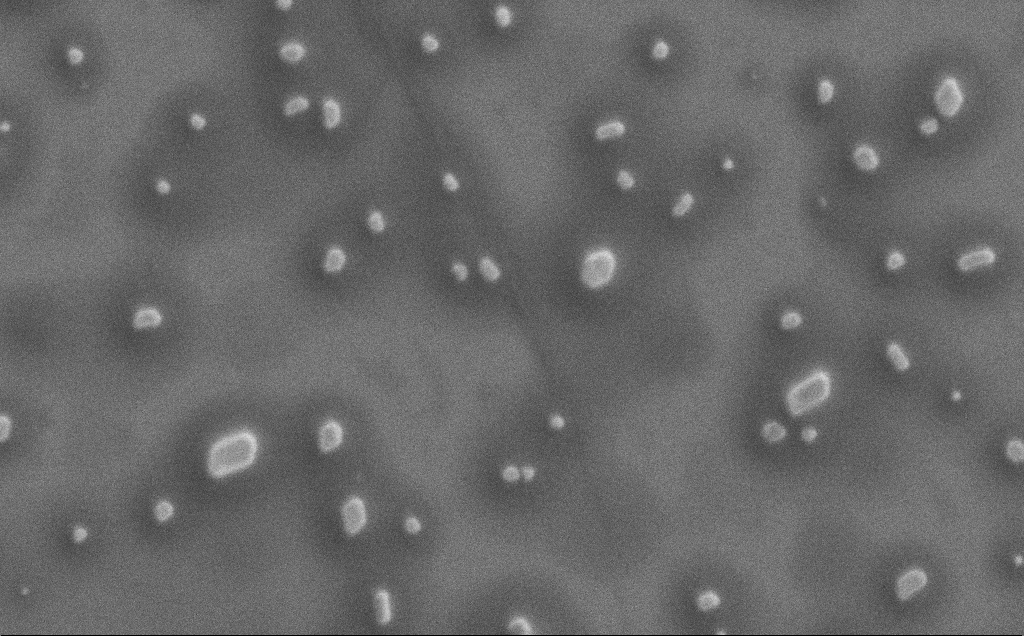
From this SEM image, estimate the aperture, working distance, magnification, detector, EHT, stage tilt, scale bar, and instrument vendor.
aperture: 30 µm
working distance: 3 mm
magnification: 20 K X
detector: SE2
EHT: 1 kV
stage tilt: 0°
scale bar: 2000 nm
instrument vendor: Zeiss